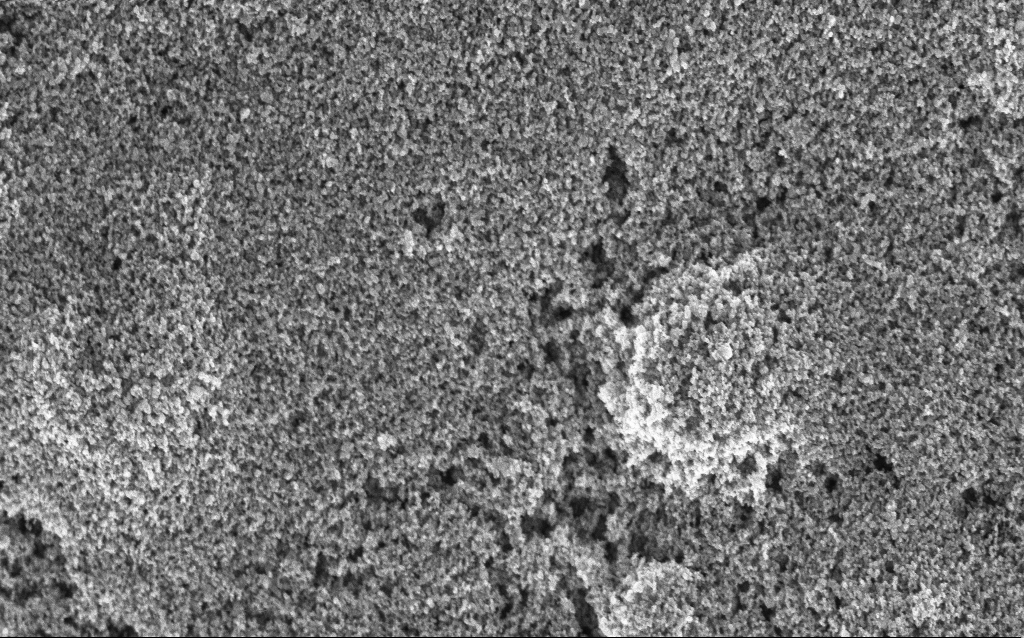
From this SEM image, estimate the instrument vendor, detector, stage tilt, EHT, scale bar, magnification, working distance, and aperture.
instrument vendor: Zeiss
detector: InLens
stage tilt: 0°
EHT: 5 kV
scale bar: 1000 nm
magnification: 37.8 K X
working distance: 2.7 mm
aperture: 30 µm